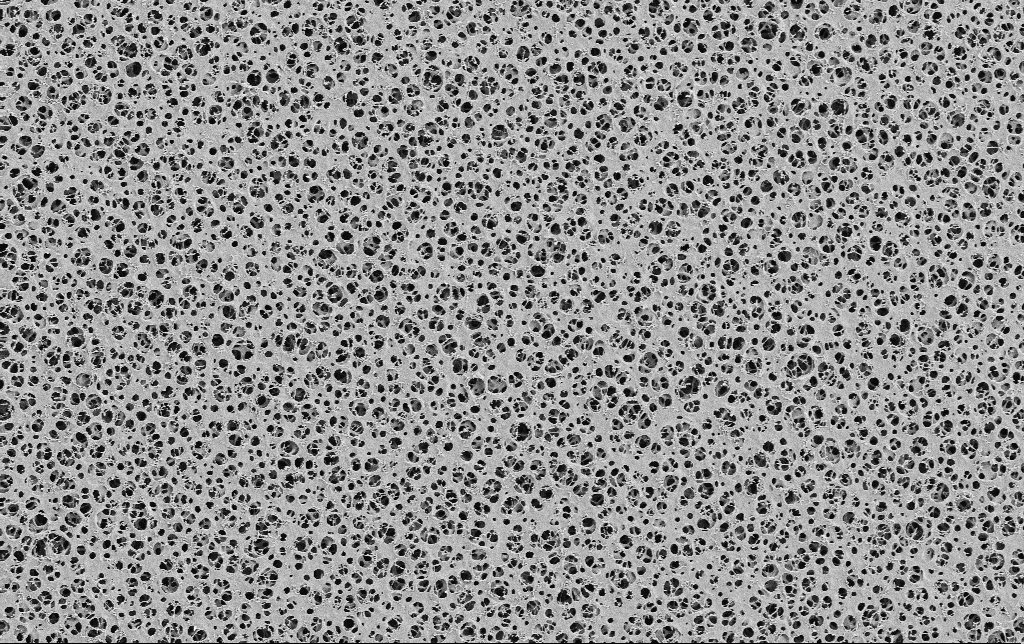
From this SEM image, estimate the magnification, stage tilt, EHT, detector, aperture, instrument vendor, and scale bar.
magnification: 2.5 K X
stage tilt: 0°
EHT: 2 kV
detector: SE2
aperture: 30 µm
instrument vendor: Zeiss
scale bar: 10000 nm